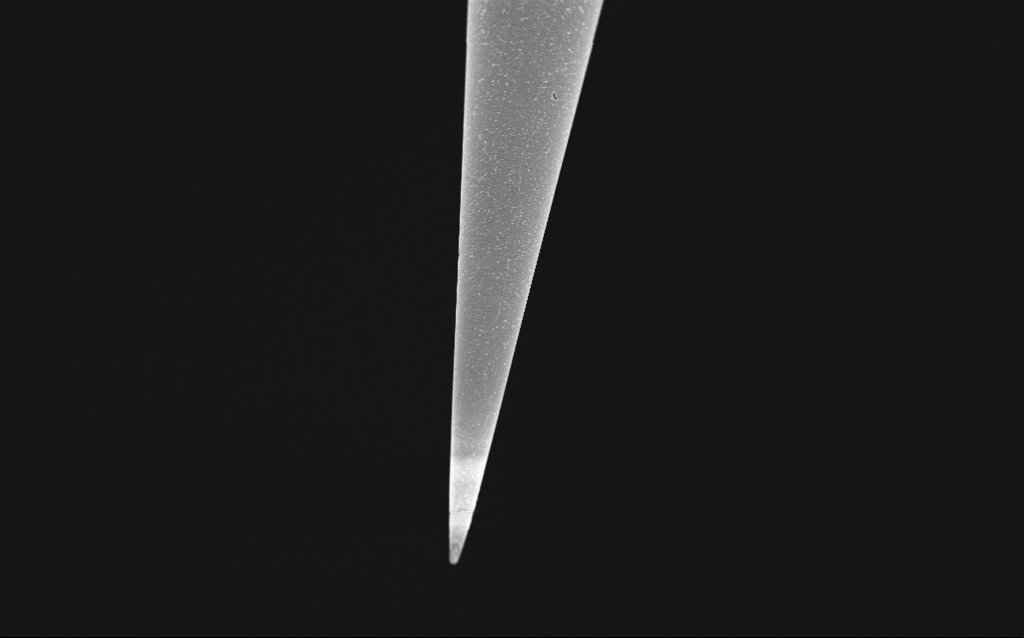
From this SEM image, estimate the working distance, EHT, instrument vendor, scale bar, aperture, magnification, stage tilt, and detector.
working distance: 6 mm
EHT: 1 kV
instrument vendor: Zeiss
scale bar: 10000 nm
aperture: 30 µm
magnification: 5 K X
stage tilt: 45°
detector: InLens